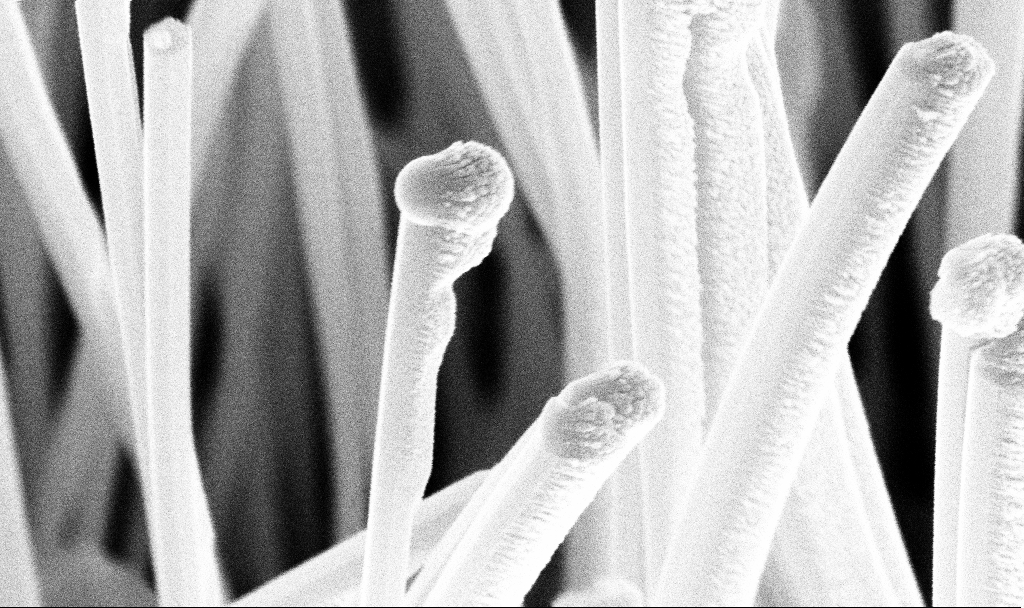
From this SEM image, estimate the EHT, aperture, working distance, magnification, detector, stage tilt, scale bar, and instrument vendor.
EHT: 10 kV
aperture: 30 µm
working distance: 7.2 mm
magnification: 150 K X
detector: InLens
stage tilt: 45°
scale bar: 200 nm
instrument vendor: Zeiss